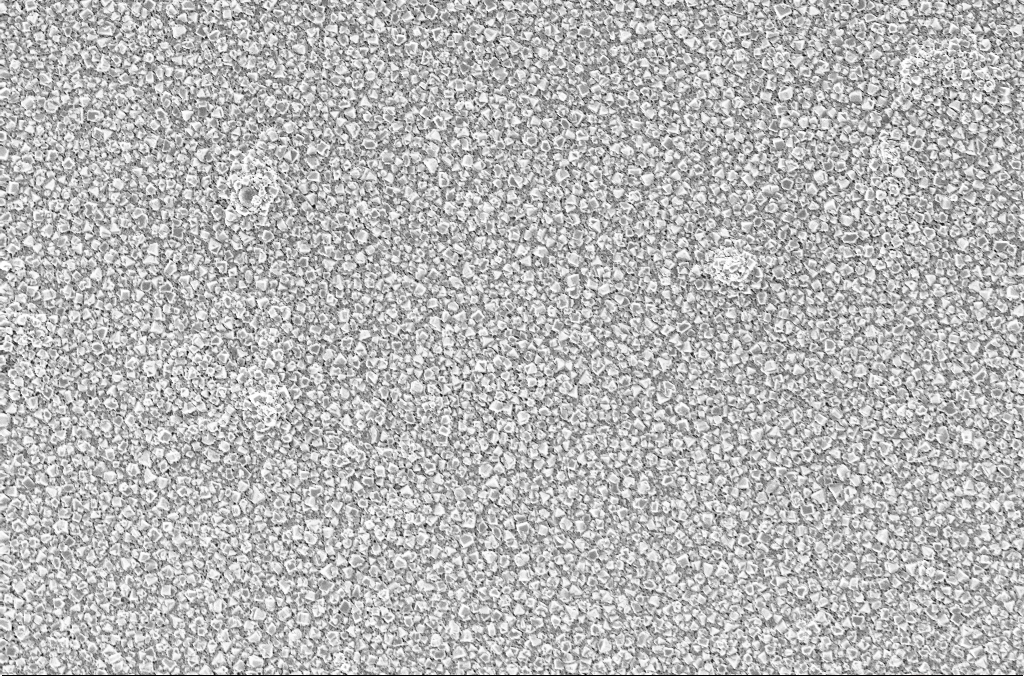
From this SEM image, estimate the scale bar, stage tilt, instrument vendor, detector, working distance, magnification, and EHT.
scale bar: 2000 nm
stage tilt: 0°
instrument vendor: Zeiss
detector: InLens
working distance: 1.9 mm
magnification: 10 K X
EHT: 2 kV